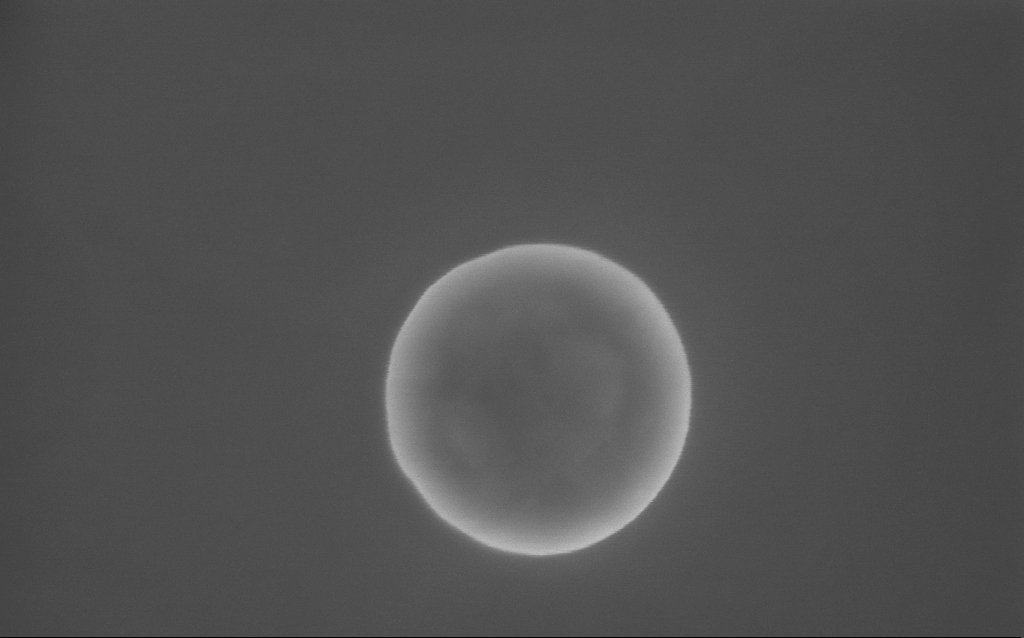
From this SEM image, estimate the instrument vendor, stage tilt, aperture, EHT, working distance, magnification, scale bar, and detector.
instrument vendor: Zeiss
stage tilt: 0°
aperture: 30 µm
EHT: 10 kV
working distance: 2 mm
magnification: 157.25 K X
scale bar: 200 nm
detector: InLens